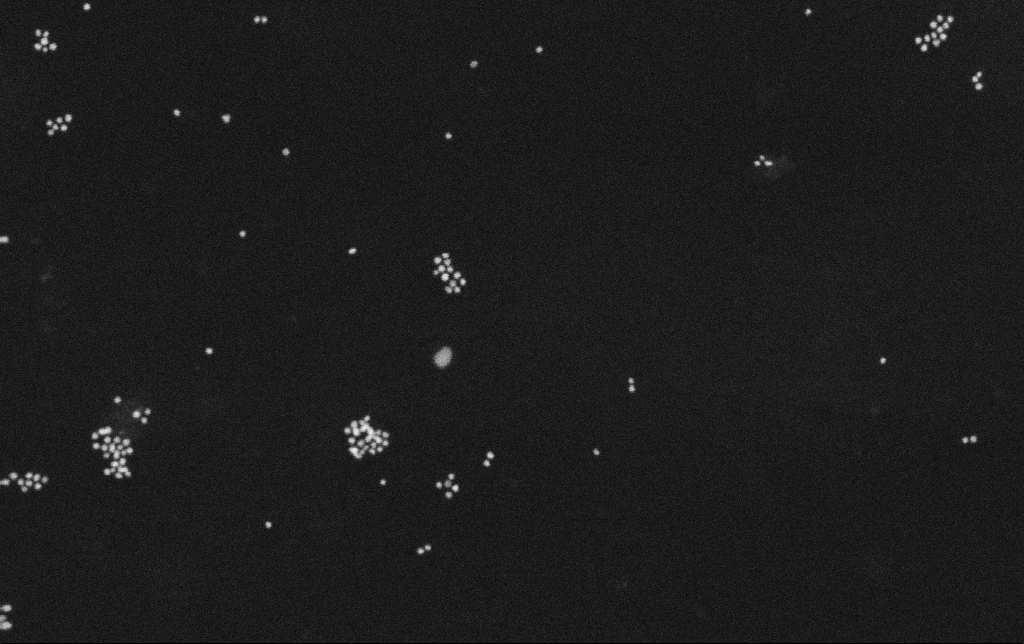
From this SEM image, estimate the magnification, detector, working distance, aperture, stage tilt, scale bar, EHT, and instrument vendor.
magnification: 200 K X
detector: SE2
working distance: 10.8 mm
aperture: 30 µm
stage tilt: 0°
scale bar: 100 nm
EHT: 30 kV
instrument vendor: Zeiss